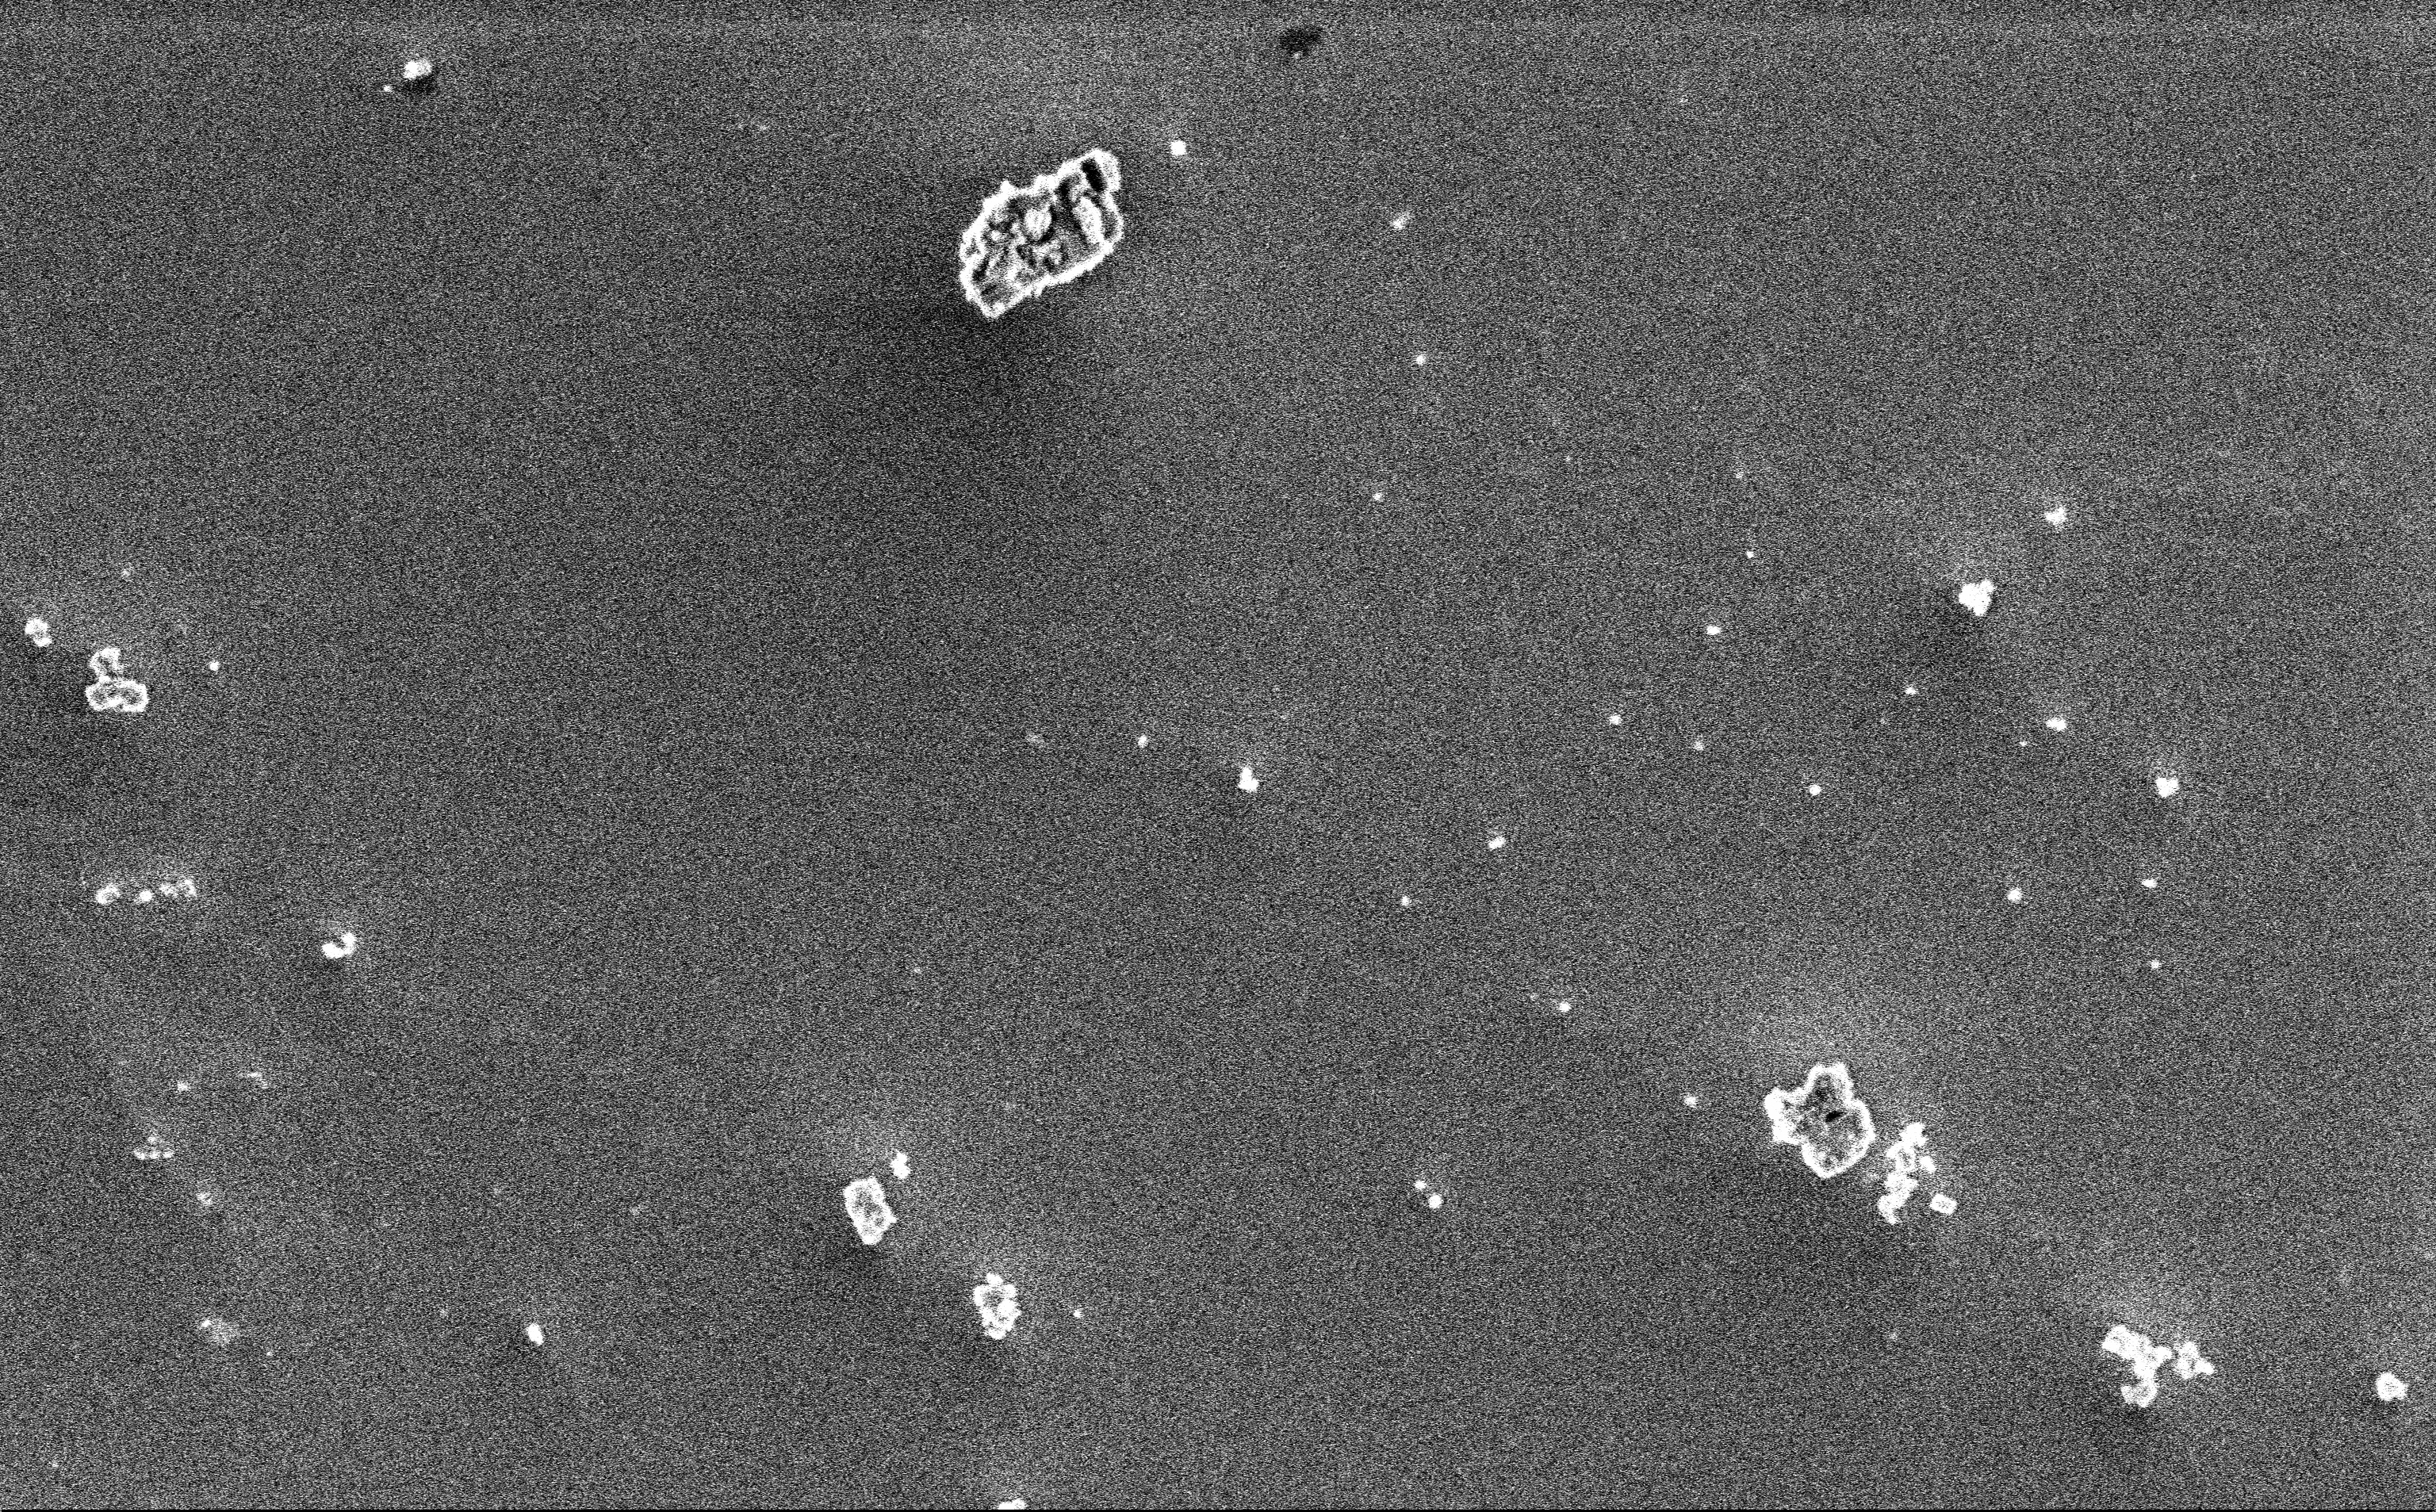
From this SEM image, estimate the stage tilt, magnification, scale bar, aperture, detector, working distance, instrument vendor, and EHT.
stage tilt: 0°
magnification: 12.85 K X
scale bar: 2000 nm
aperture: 30 µm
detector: InLens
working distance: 3 mm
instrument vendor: Zeiss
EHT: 3 kV